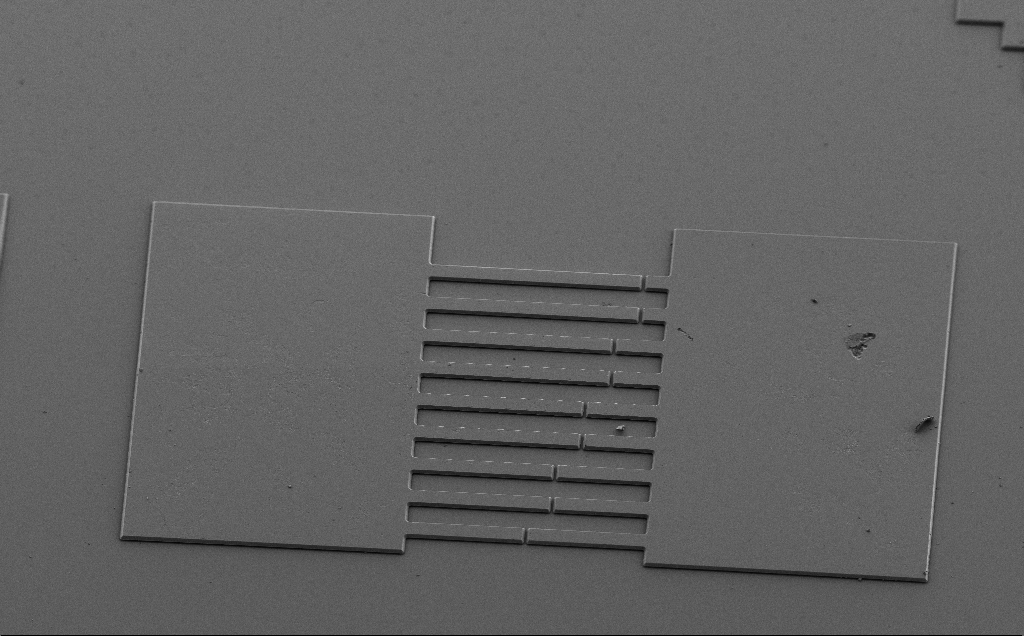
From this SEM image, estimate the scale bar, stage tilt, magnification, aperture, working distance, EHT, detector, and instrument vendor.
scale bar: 100000 nm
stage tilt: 30°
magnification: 0.35 K X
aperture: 30 µm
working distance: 6 mm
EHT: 3 kV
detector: SE2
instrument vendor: Zeiss